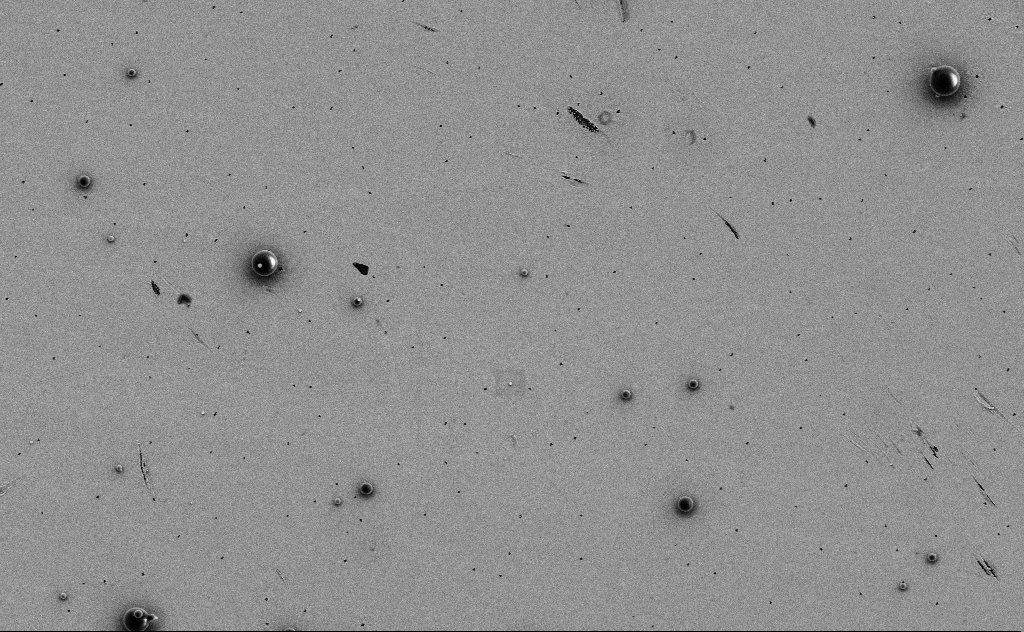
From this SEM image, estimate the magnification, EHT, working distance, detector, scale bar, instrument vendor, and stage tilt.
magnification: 6.87 K X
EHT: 3 kV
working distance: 10 mm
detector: SE2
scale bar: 10000 nm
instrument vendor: Zeiss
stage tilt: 0°